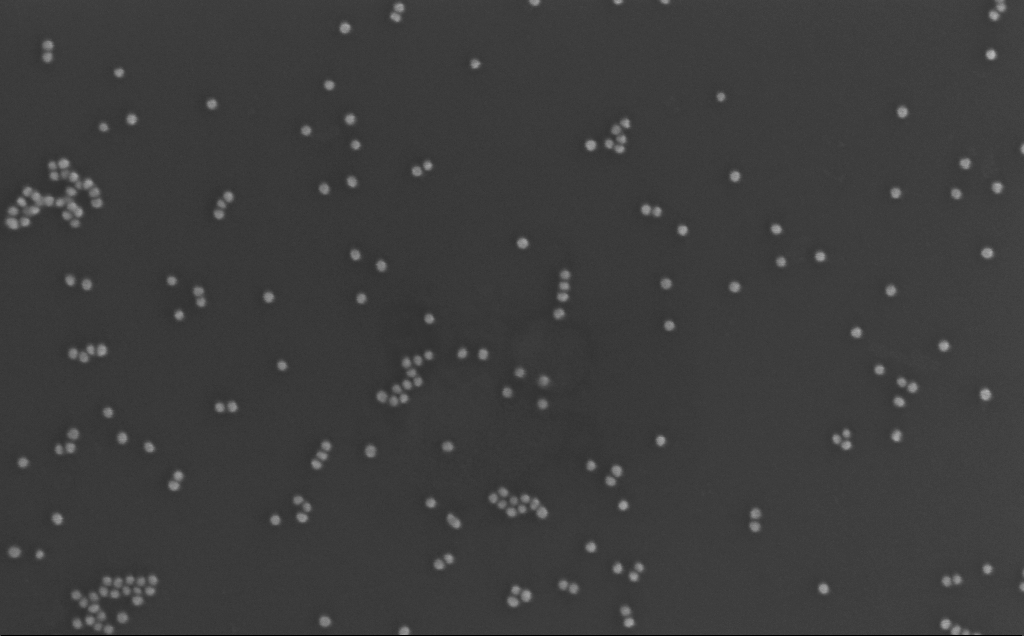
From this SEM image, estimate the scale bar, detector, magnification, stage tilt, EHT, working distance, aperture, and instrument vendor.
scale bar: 200 nm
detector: InLens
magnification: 222.33 K X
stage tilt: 0°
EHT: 10 kV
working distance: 3 mm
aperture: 30 µm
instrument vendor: Zeiss